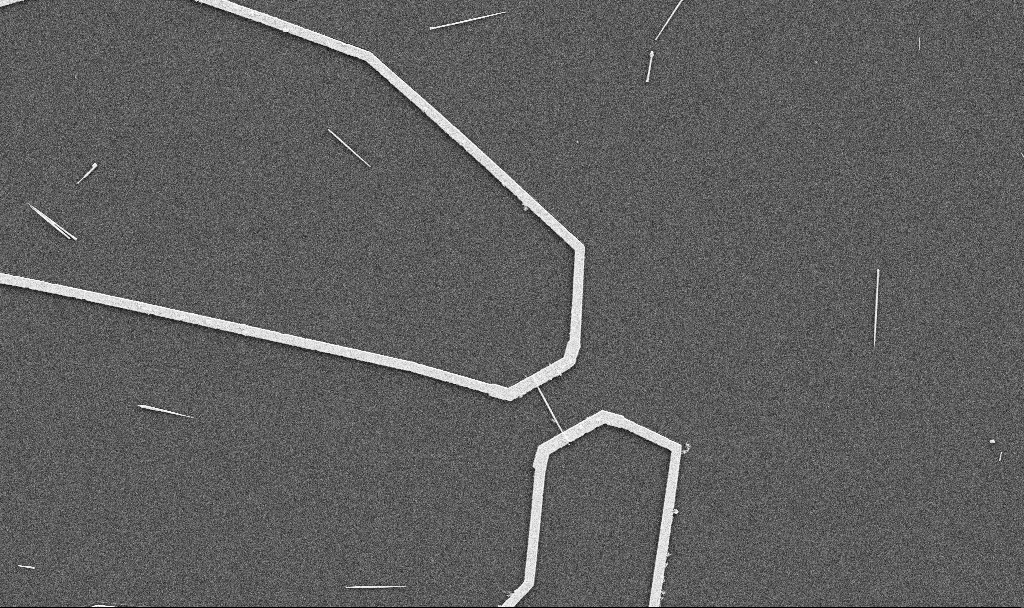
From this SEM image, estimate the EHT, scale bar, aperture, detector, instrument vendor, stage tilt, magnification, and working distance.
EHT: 5 kV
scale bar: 10000 nm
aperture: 30 µm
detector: SE2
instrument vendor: Zeiss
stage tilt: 0°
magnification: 5 K X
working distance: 10.7 mm